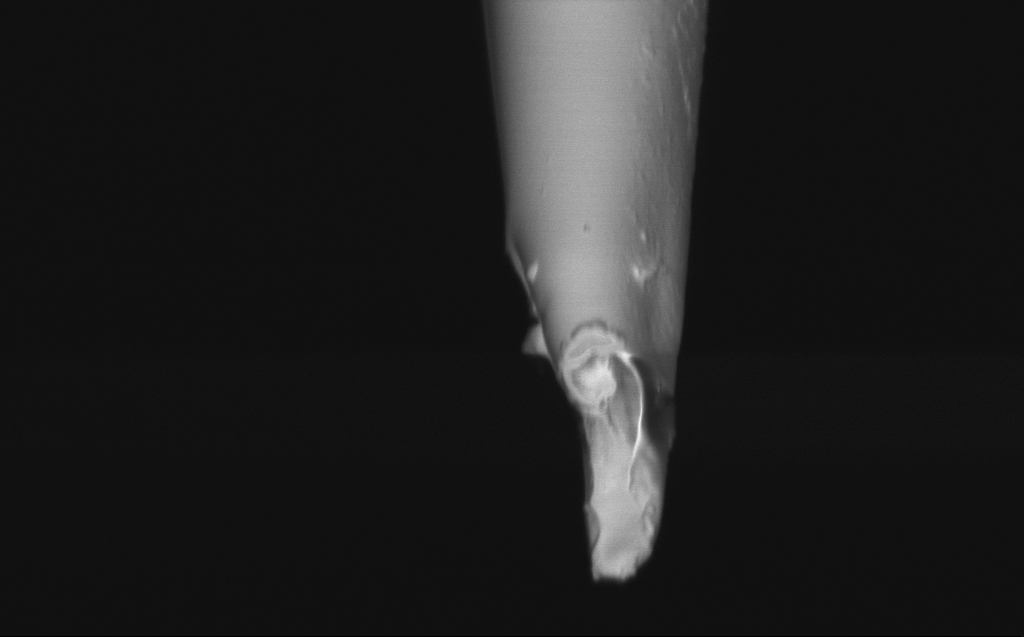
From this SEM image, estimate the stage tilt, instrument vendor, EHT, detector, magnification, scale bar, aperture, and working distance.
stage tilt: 45°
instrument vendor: Zeiss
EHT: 1 kV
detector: InLens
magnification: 50 K X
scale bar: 1000 nm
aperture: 30 µm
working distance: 5 mm